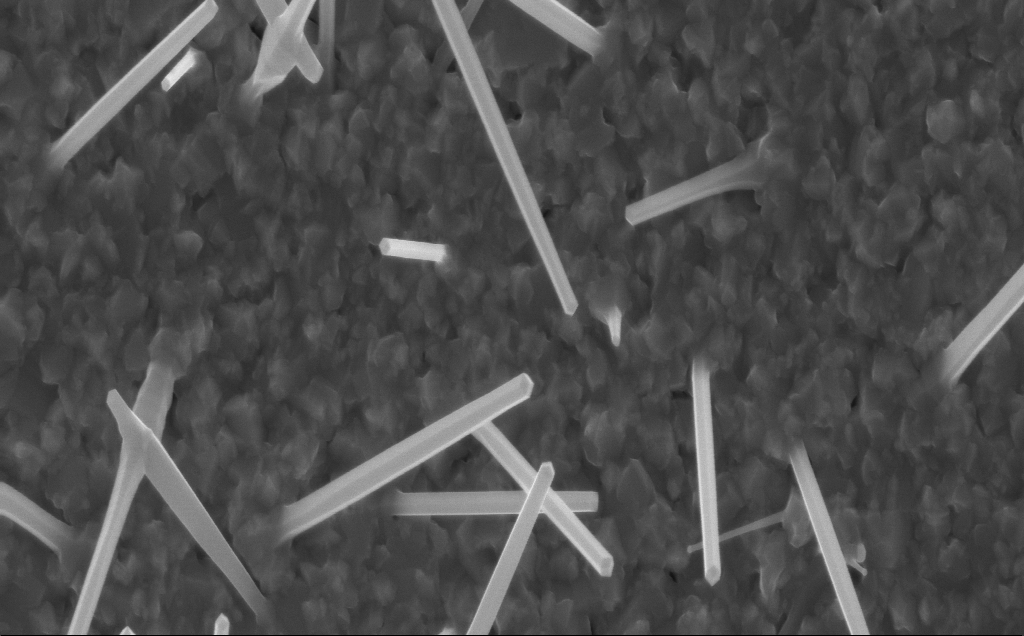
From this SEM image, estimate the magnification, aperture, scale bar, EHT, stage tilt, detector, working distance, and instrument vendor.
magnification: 31.72 K X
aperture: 30 µm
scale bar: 2000 nm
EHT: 10 kV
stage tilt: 0°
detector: InLens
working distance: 4 mm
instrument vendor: Zeiss